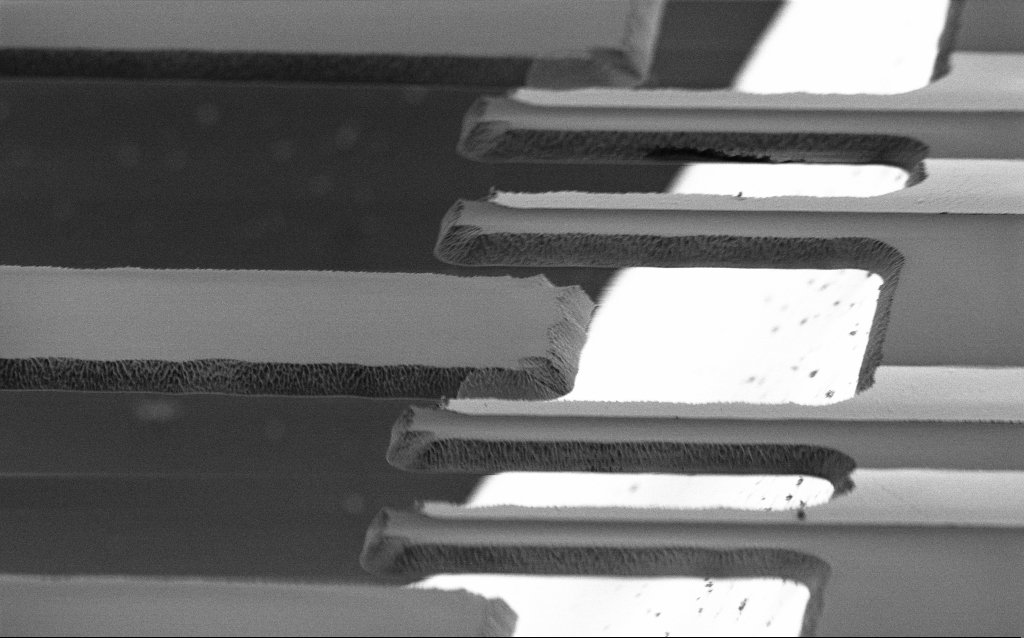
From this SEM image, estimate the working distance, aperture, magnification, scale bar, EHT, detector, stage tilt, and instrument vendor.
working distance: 8.9 mm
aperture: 30 µm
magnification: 5.52 K X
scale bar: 10000 nm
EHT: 2 kV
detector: SE2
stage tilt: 70°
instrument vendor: Zeiss